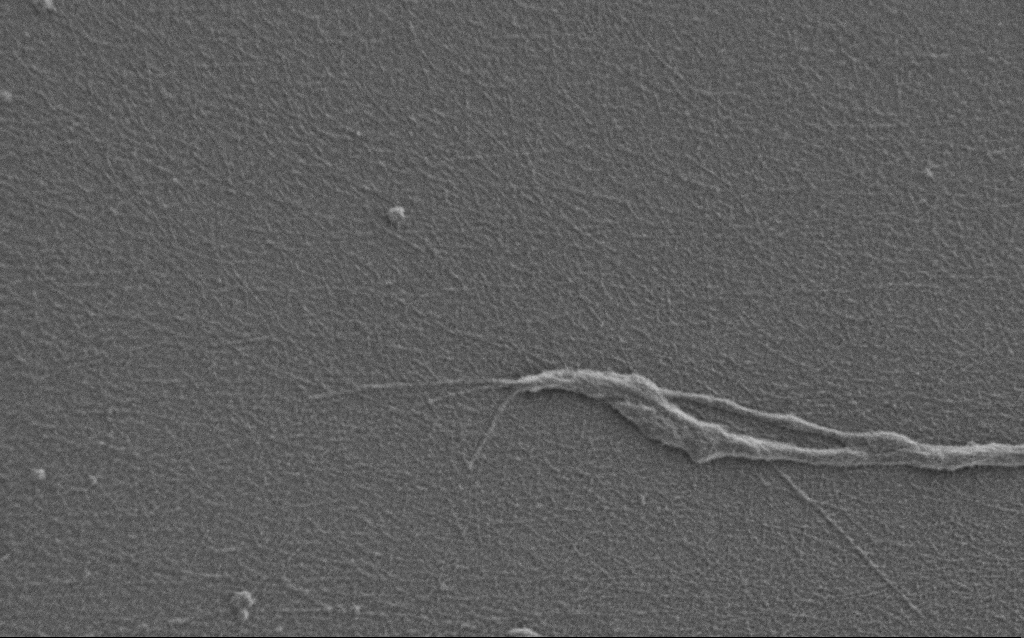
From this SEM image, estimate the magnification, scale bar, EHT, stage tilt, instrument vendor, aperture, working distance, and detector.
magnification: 10 K X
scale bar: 2000 nm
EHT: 0.9 kV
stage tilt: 0°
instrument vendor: Zeiss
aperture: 30 µm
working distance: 7 mm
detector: SE2